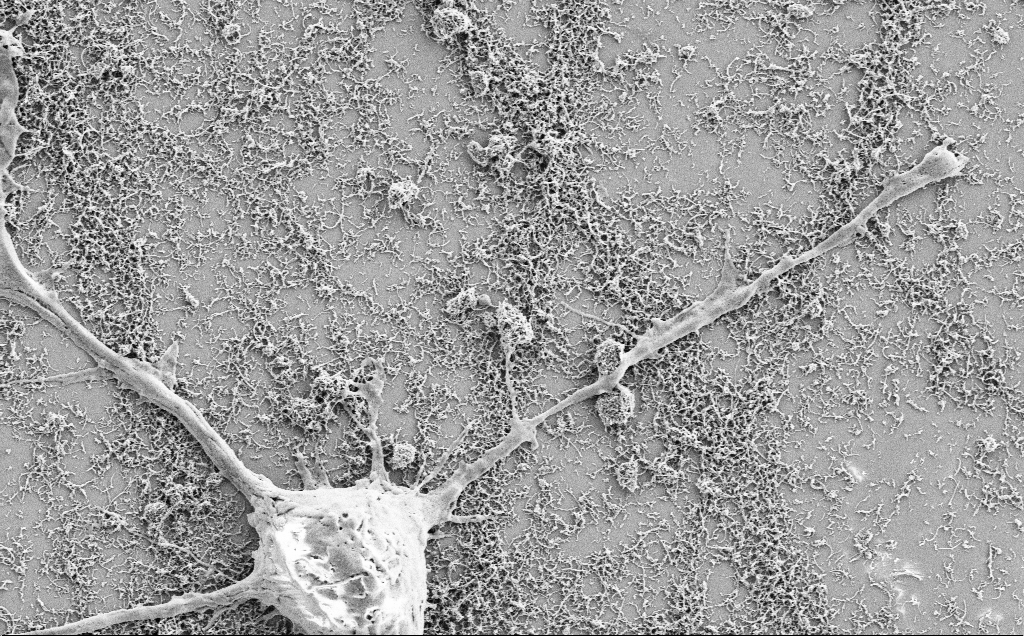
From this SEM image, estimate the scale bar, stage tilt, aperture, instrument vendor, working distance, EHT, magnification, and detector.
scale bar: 2000 nm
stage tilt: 0°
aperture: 30 µm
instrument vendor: Zeiss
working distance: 7.1 mm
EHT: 2 kV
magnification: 10 K X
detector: SE2